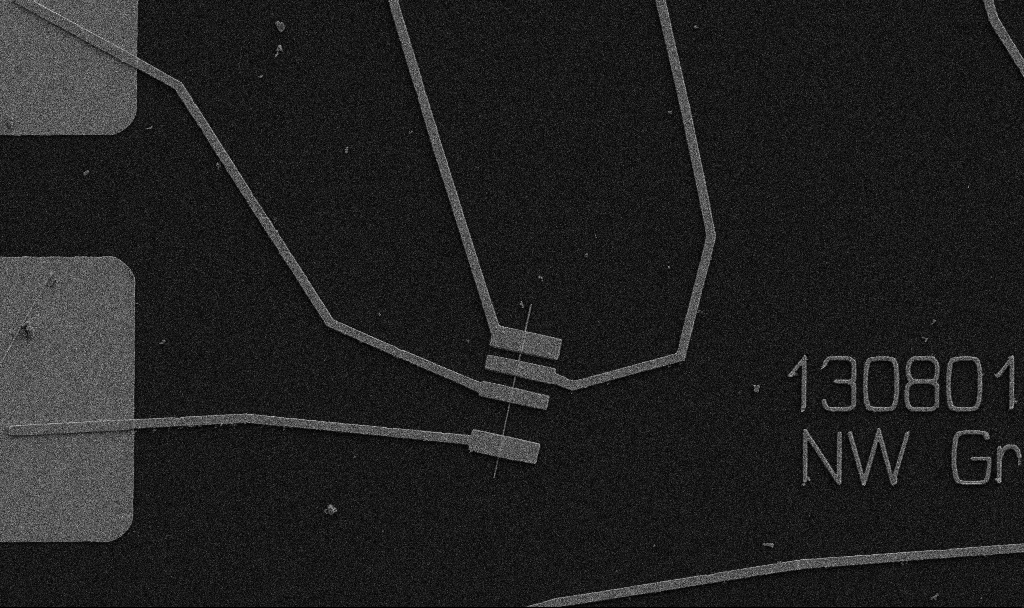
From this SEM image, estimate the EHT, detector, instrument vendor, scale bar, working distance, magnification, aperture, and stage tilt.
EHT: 5 kV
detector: SE2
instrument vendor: Zeiss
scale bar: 10000 nm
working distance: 10.7 mm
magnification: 5 K X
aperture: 30 µm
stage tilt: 0°